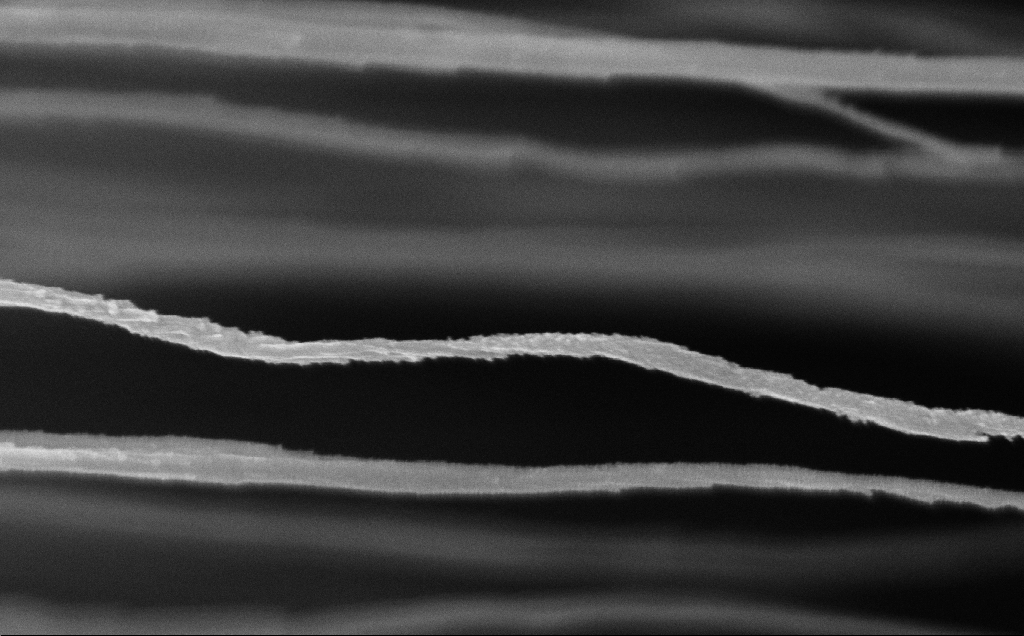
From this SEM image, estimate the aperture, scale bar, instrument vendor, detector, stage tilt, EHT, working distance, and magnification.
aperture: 30 µm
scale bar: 200 nm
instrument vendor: Zeiss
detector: InLens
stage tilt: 0°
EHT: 5 kV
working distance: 12 mm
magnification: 101.46 K X